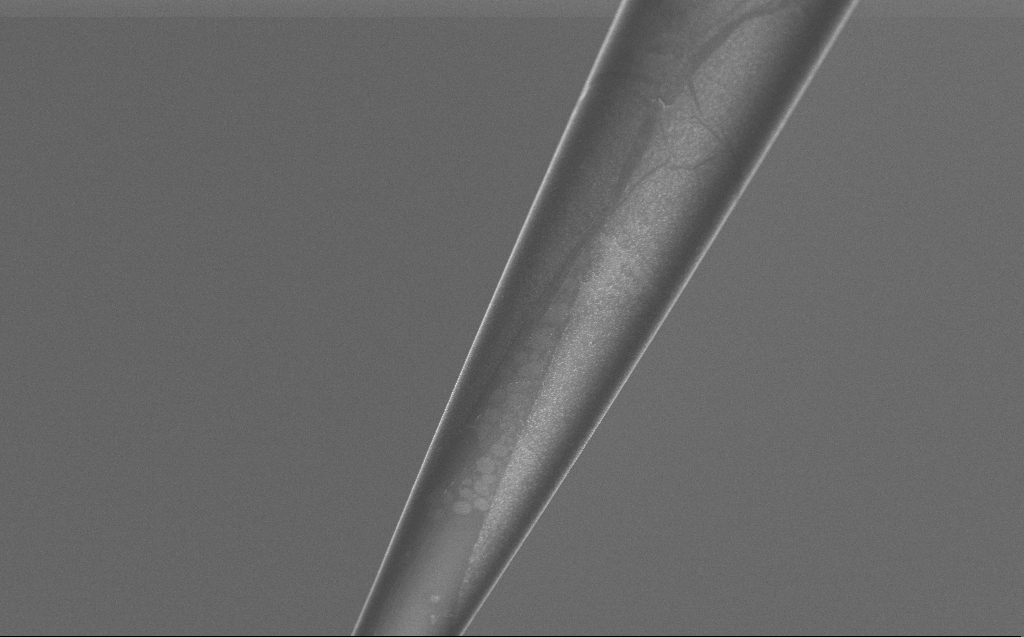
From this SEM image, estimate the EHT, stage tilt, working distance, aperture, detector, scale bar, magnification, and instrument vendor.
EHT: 5 kV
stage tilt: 45°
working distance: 6 mm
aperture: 30 µm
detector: InLens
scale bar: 10000 nm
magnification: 5 K X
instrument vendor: Zeiss